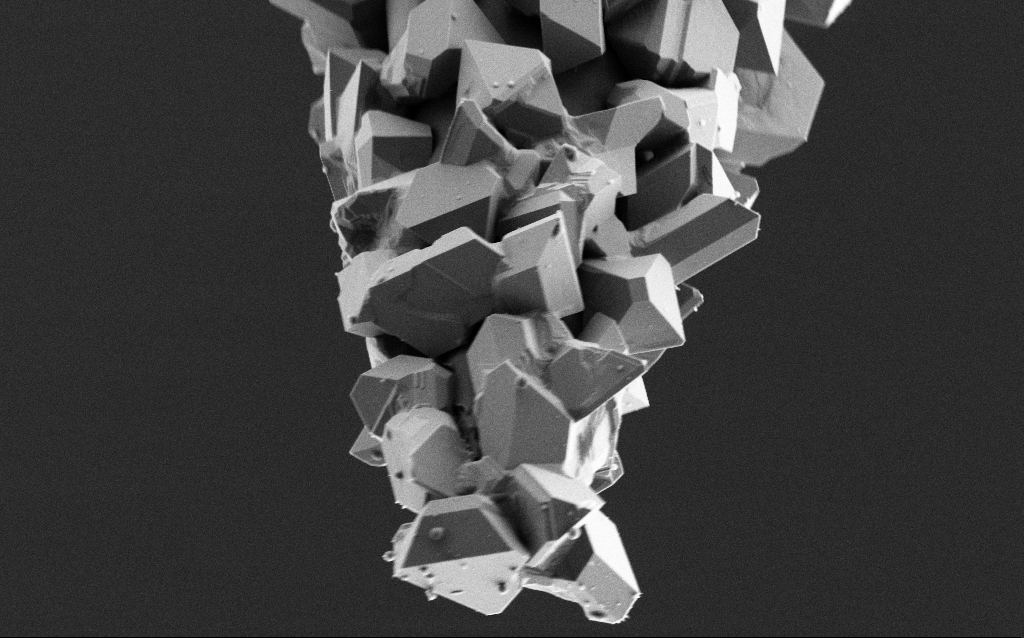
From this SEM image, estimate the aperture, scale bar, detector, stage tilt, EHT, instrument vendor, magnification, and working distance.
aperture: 30 µm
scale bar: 1000 nm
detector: SE2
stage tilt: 45°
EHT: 2 kV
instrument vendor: Zeiss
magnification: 20 K X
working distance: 4 mm